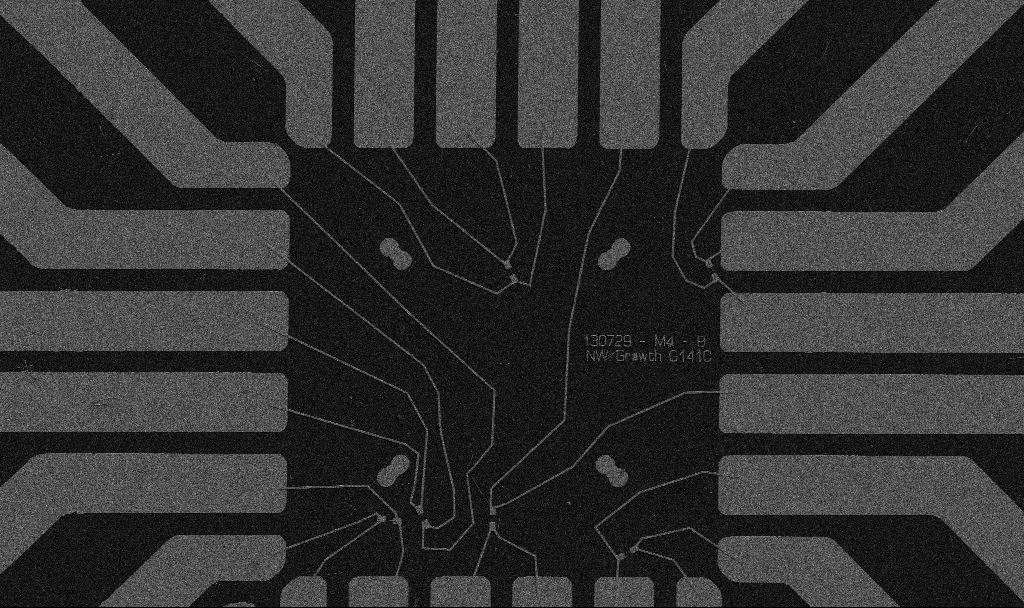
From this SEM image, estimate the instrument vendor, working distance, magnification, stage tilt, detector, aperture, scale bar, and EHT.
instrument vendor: Zeiss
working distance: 10.7 mm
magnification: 1 K X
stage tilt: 0°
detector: SE2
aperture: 30 µm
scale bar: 20000 nm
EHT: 5 kV